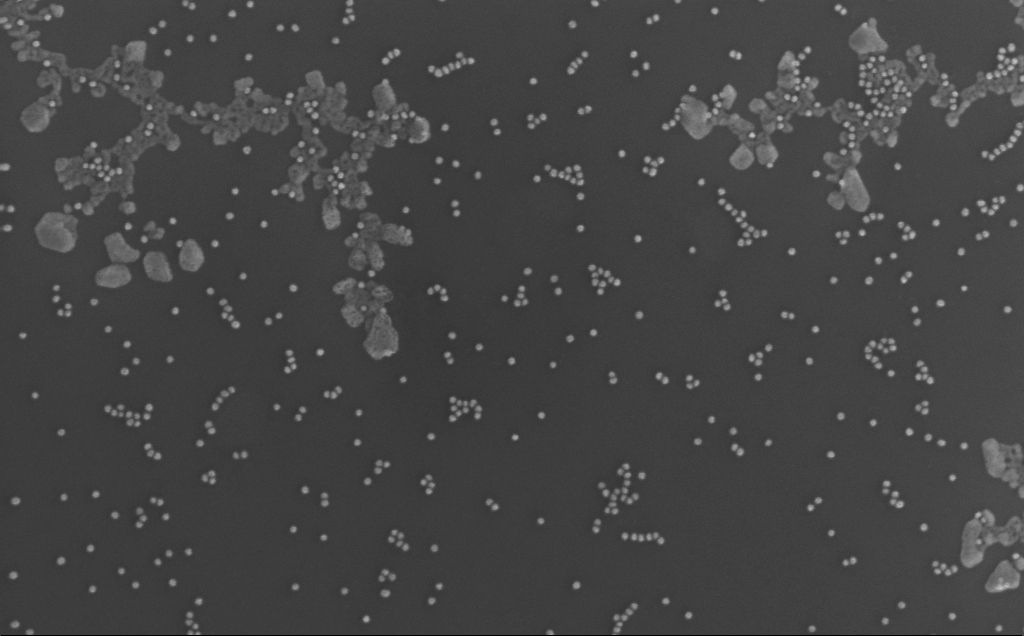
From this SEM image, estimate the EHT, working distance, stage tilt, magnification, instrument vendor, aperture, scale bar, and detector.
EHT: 10 kV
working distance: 3 mm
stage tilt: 0°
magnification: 147.7 K X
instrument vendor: Zeiss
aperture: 30 µm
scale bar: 200 nm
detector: InLens